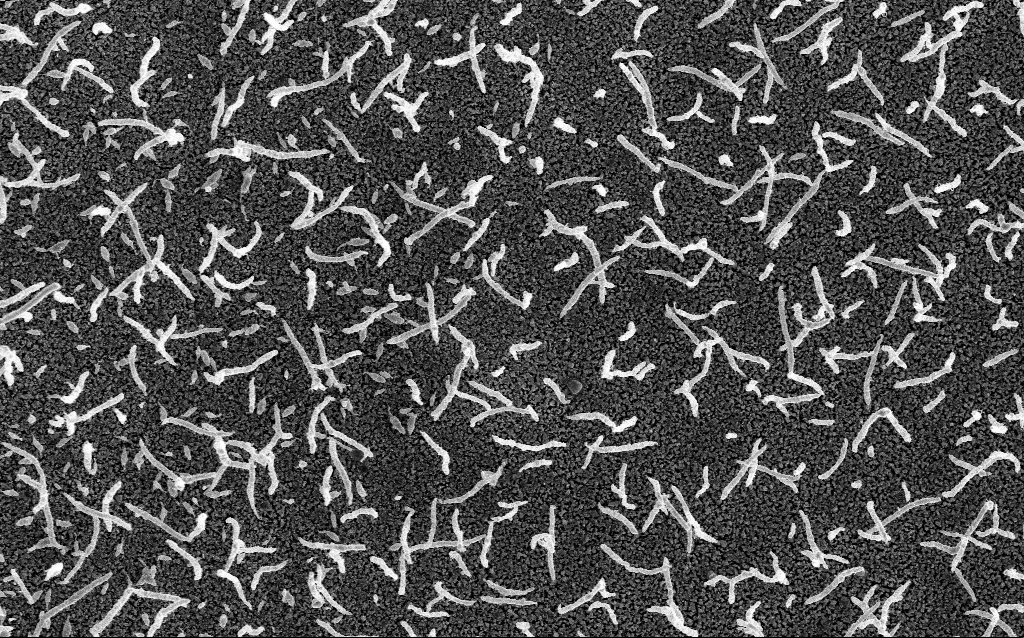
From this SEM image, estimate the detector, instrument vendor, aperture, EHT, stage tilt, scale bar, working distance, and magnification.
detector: InLens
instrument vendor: Zeiss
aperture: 30 µm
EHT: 5 kV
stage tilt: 0°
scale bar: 1000 nm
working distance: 1.8 mm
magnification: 20 K X